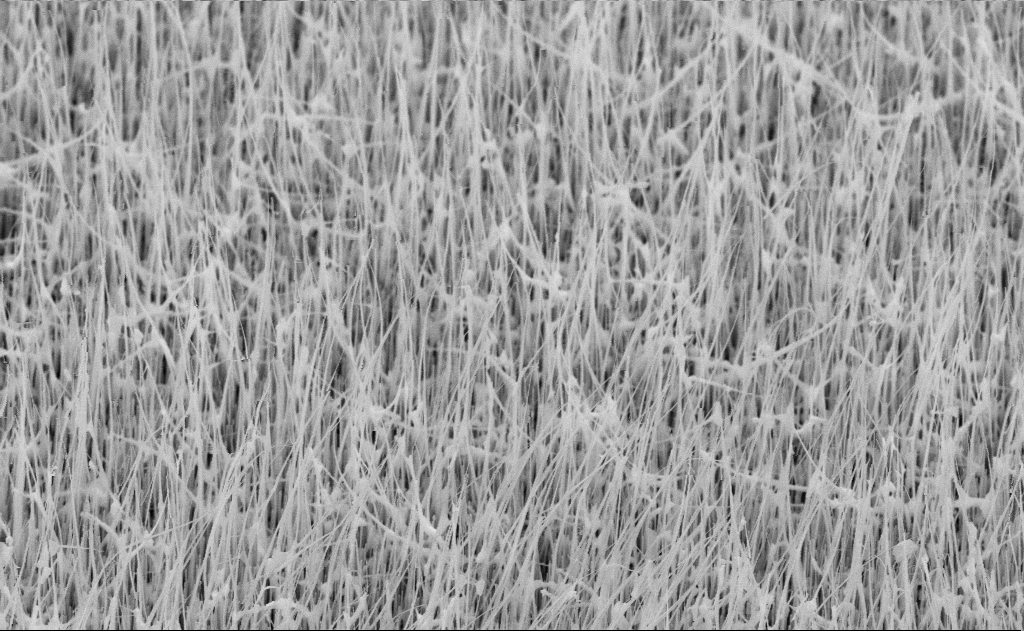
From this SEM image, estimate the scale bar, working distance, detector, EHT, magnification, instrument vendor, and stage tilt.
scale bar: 1000 nm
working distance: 15 mm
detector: SE2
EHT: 10 kV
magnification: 20 K X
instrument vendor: Zeiss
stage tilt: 45°